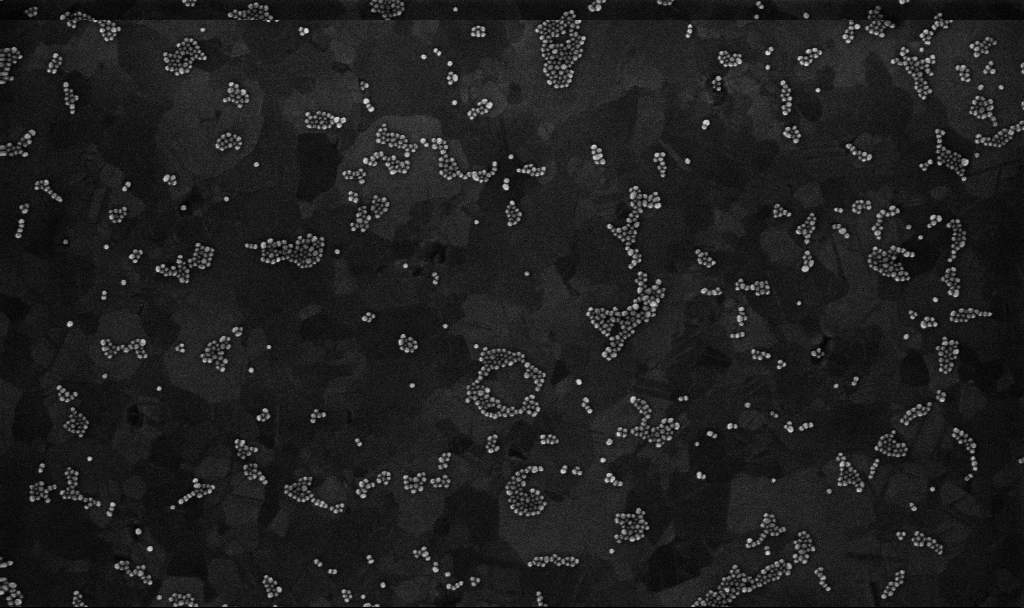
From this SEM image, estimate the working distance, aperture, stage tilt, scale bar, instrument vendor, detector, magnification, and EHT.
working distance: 3.3 mm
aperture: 30 µm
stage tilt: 0°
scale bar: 200 nm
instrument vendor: Zeiss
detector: InLens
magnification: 100 K X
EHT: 10 kV